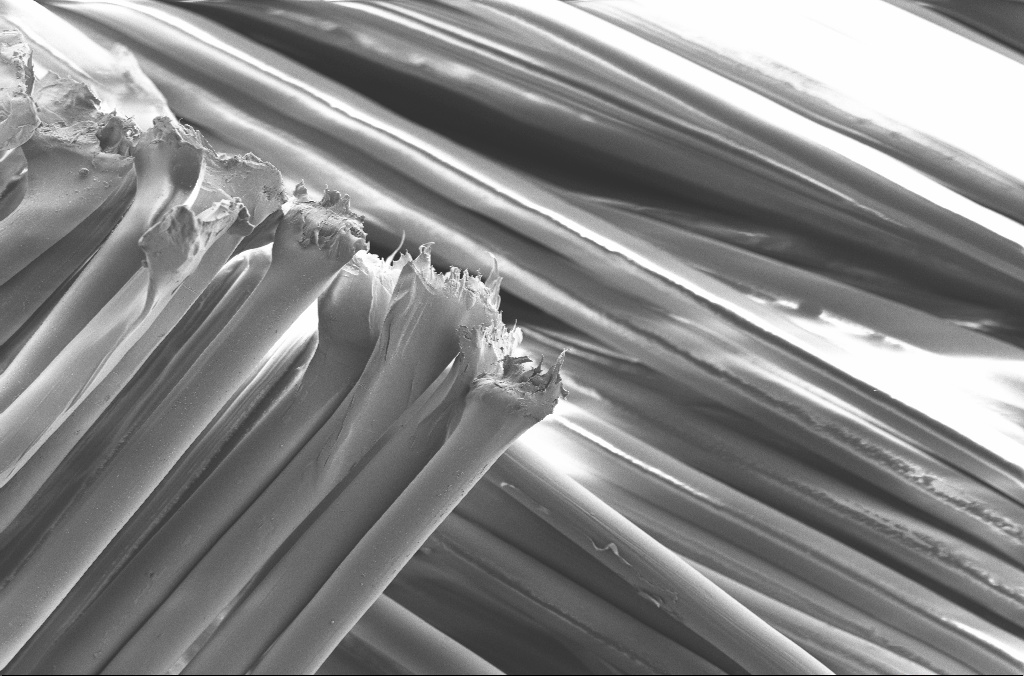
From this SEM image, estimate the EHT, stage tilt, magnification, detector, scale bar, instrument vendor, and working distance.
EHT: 1.5 kV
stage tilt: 0°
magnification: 1 K X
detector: SE2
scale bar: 20000 nm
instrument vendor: Zeiss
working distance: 5.4 mm